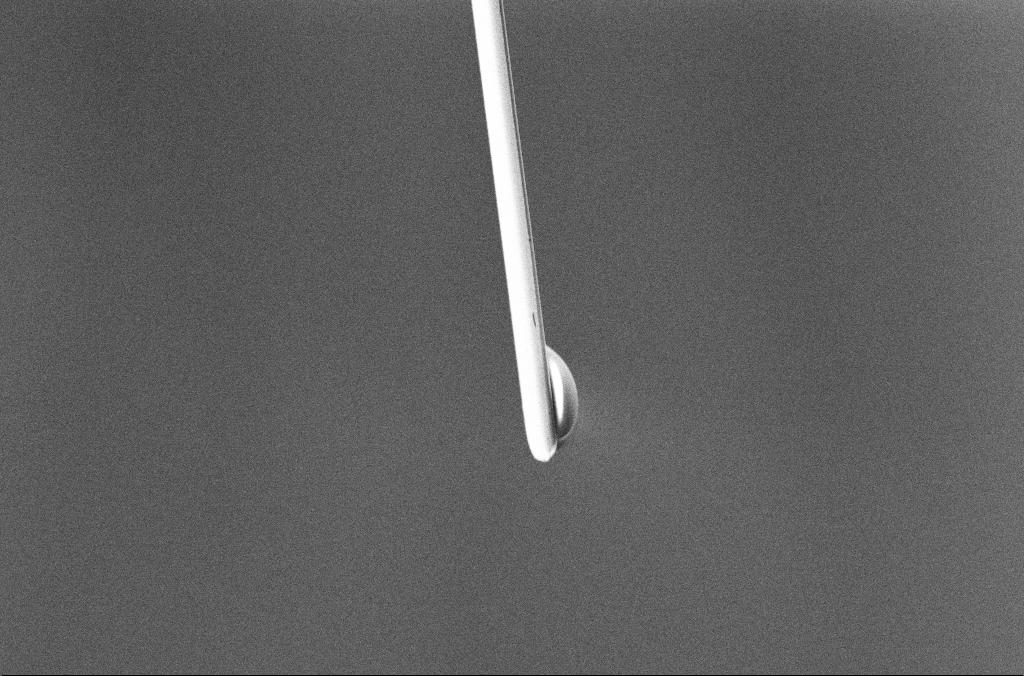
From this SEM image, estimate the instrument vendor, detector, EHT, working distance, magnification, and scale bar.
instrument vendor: Zeiss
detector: InLens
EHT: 5 kV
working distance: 5.3 mm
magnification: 2.5 K X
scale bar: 10000 nm